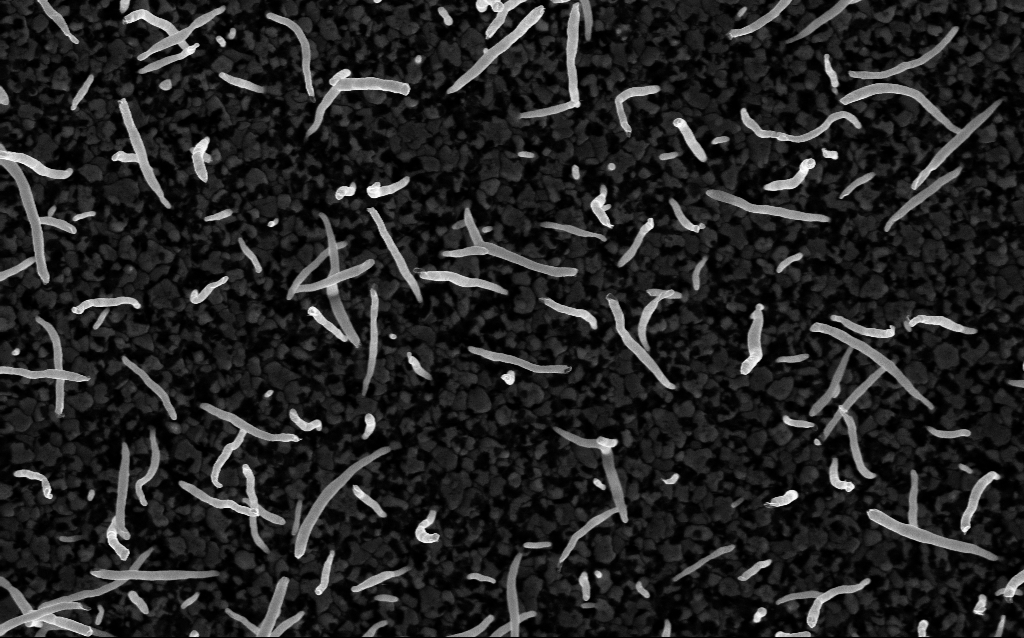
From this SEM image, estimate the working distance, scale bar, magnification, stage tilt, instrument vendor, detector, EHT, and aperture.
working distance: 2.6 mm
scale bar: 1000 nm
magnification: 50 K X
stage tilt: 0°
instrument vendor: Zeiss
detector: InLens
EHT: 5 kV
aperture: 30 µm